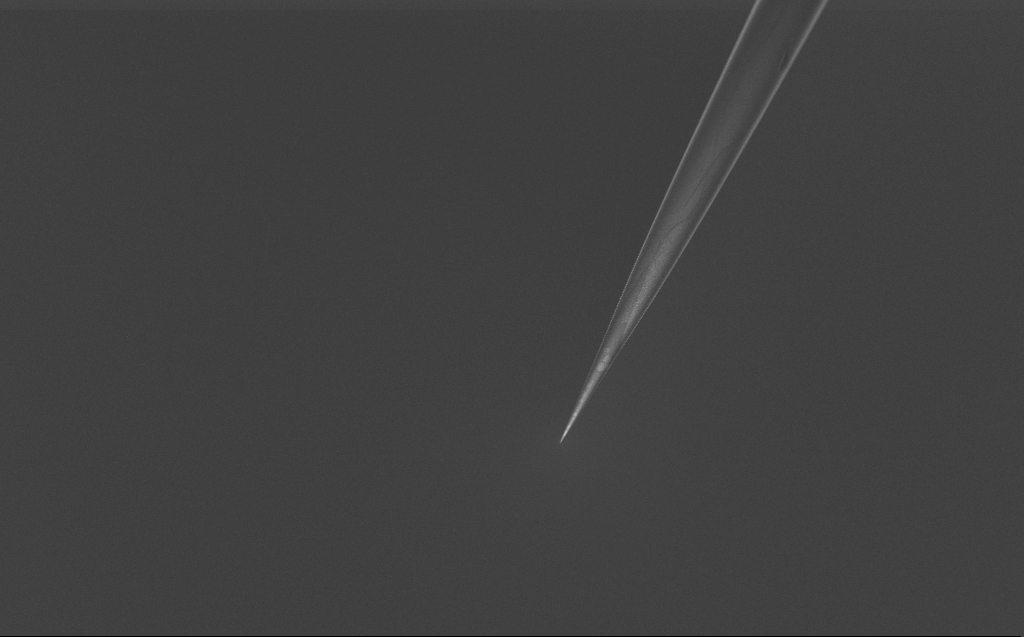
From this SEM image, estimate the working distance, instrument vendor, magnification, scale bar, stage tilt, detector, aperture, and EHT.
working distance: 6 mm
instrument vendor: Zeiss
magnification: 1.01 K X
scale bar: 20000 nm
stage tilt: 45°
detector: InLens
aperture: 30 µm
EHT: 2 kV